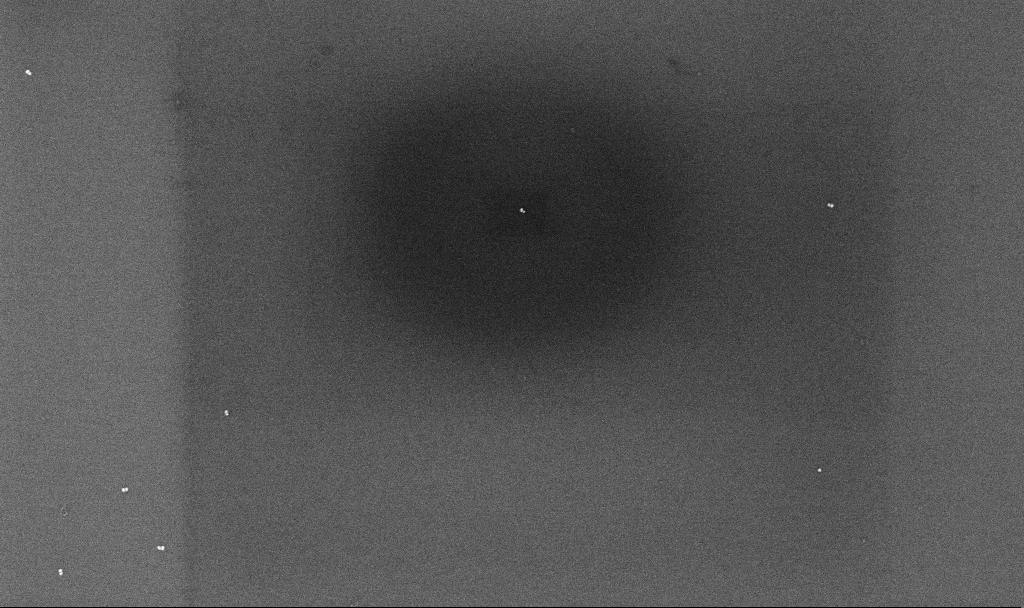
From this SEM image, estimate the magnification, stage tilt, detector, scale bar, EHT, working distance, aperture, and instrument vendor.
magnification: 68.78 K X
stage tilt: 0°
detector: InLens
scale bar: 1000 nm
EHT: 10 kV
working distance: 4.3 mm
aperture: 30 µm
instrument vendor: Zeiss